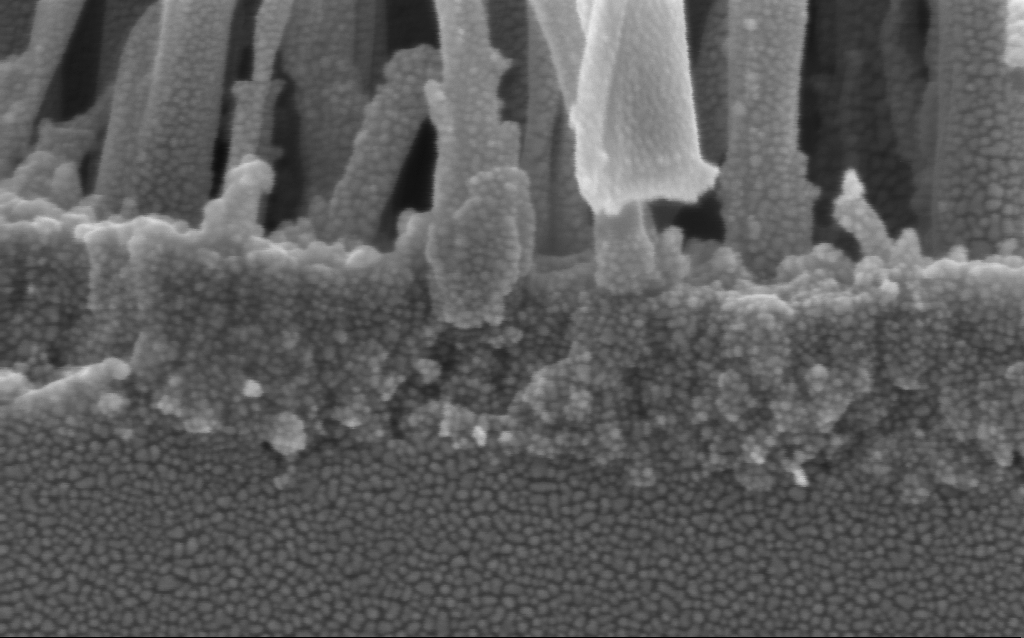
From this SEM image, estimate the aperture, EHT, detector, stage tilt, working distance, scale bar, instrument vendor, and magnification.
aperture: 30 µm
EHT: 3 kV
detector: InLens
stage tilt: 0°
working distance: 3 mm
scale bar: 200 nm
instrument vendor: Zeiss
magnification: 228.49 K X